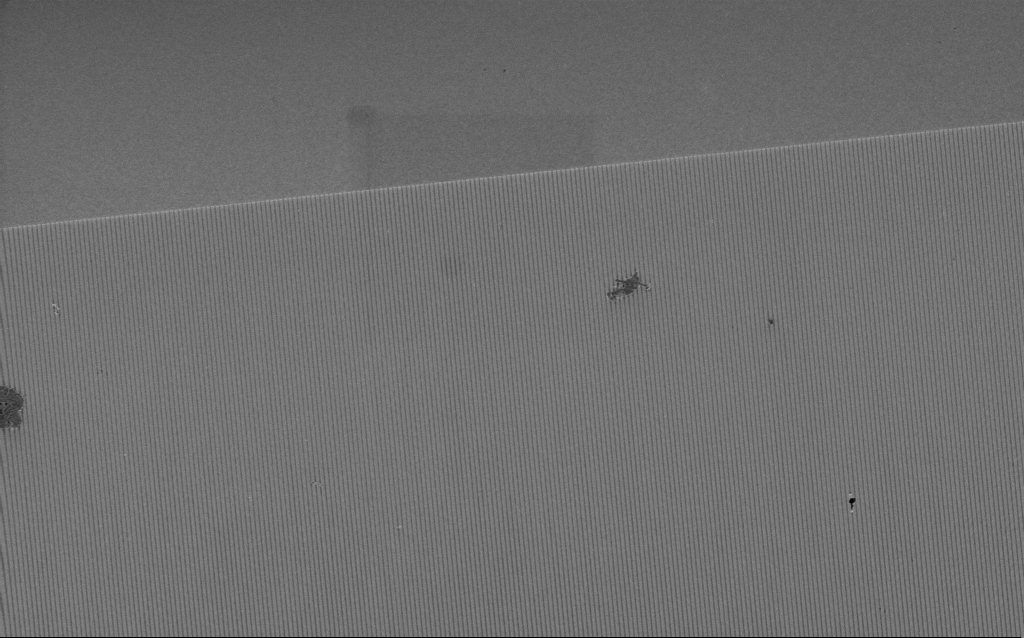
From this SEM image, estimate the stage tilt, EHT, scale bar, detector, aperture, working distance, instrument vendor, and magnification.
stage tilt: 0°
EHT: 3 kV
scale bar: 20000 nm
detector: InLens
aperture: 30 µm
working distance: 5.7 mm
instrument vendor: Zeiss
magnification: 2.76 K X